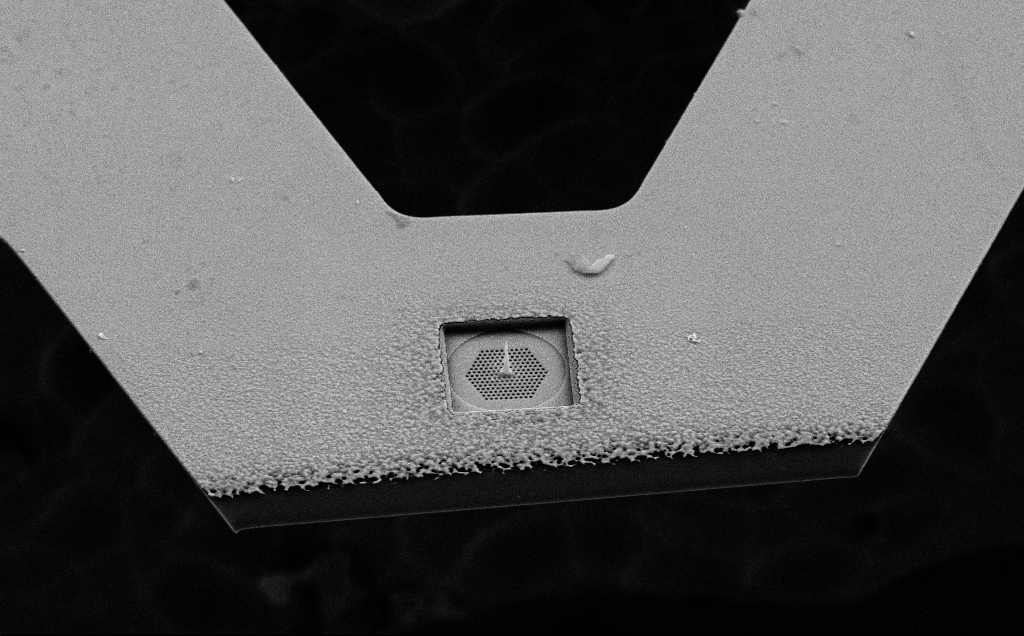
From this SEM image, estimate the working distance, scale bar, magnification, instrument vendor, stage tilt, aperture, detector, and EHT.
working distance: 8 mm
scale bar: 2000 nm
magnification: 7.3 K X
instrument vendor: Zeiss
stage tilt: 45°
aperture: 30 µm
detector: SE2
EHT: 10 kV